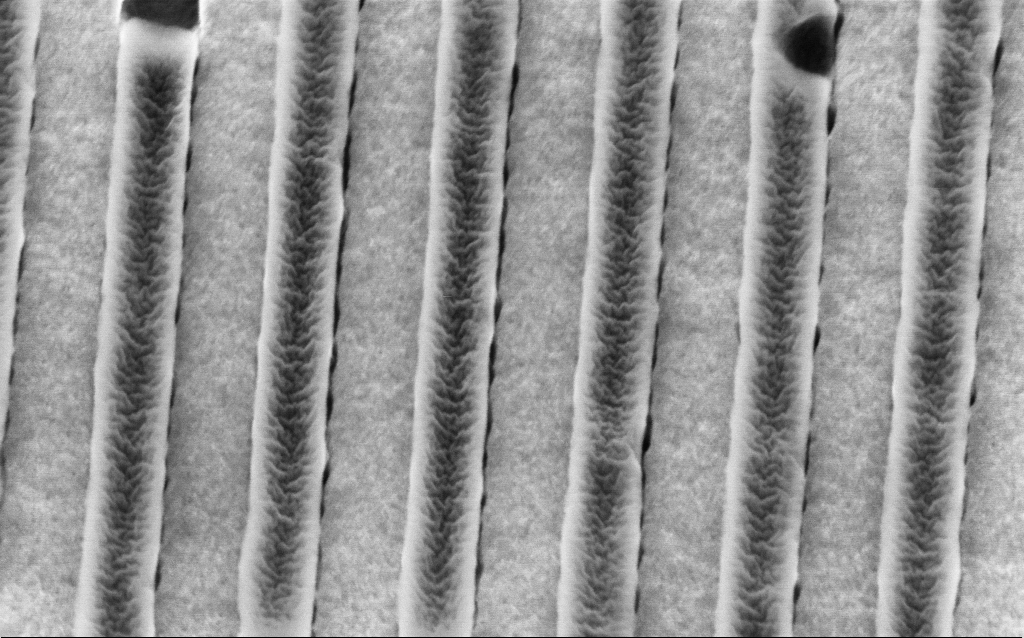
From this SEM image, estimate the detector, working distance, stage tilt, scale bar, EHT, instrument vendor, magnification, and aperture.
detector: InLens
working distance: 4.2 mm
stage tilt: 32°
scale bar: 200 nm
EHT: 2 kV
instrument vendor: Zeiss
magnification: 117.72 K X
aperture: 30 µm